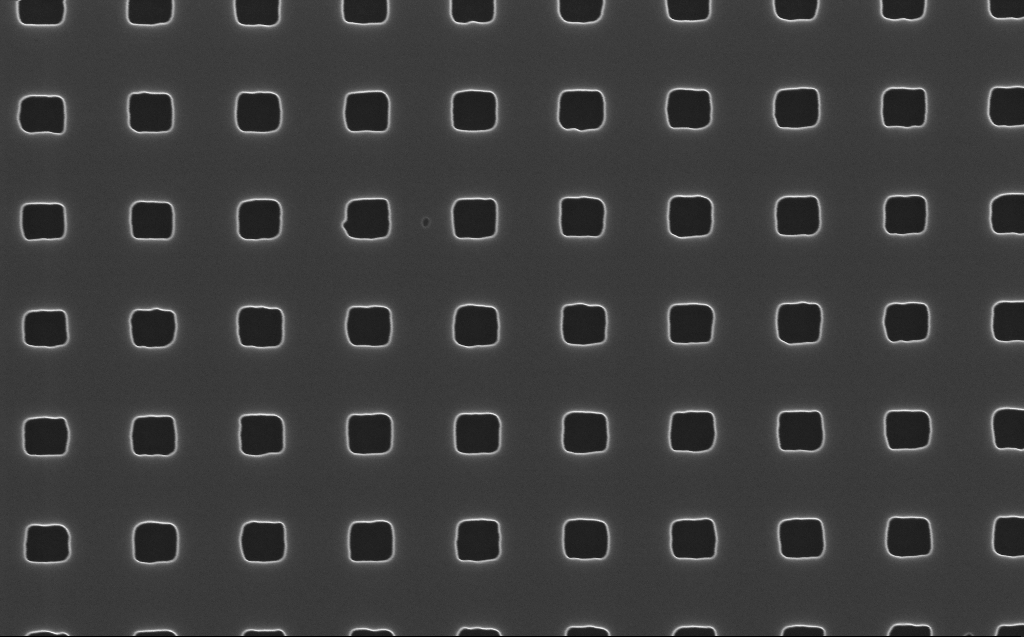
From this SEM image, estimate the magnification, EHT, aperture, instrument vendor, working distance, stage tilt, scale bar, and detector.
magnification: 80 K X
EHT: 10 kV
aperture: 30 µm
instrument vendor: Zeiss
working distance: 6 mm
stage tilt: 0°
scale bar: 200 nm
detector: InLens